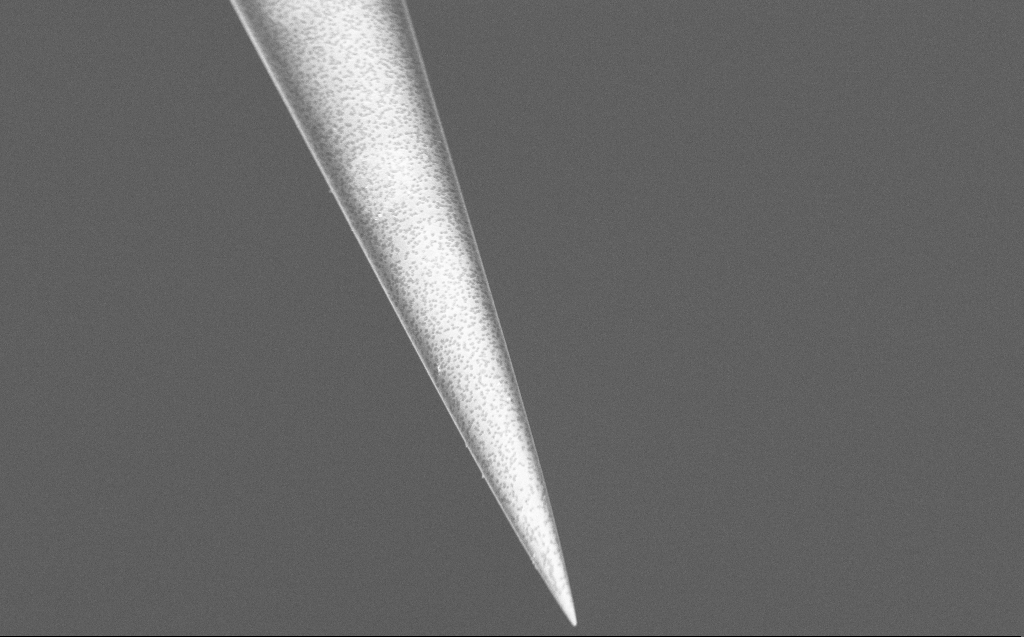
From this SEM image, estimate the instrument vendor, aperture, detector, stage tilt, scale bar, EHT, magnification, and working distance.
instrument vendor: Zeiss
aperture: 30 µm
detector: InLens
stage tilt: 45°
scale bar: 2000 nm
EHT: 5 kV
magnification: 10 K X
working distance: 7 mm